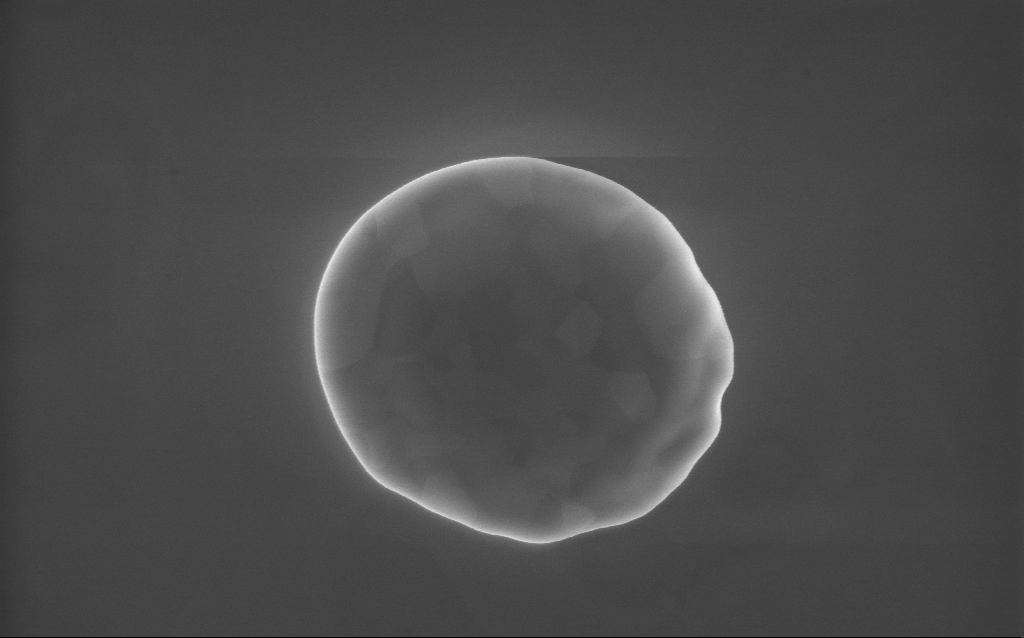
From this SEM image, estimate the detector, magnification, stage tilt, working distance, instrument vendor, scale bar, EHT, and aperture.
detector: InLens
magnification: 63 K X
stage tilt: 0°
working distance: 2 mm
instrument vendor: Zeiss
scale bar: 1000 nm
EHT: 10 kV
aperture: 30 µm